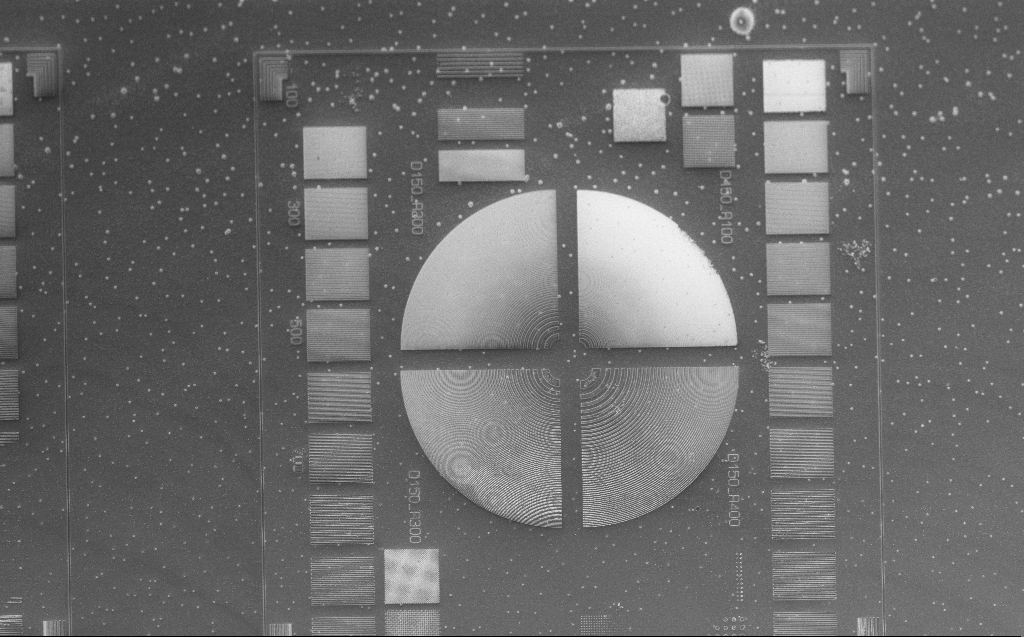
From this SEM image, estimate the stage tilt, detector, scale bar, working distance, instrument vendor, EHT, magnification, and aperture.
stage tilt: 30°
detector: InLens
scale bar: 20000 nm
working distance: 6 mm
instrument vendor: Zeiss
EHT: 3 kV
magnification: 0.776 K X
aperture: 30 µm